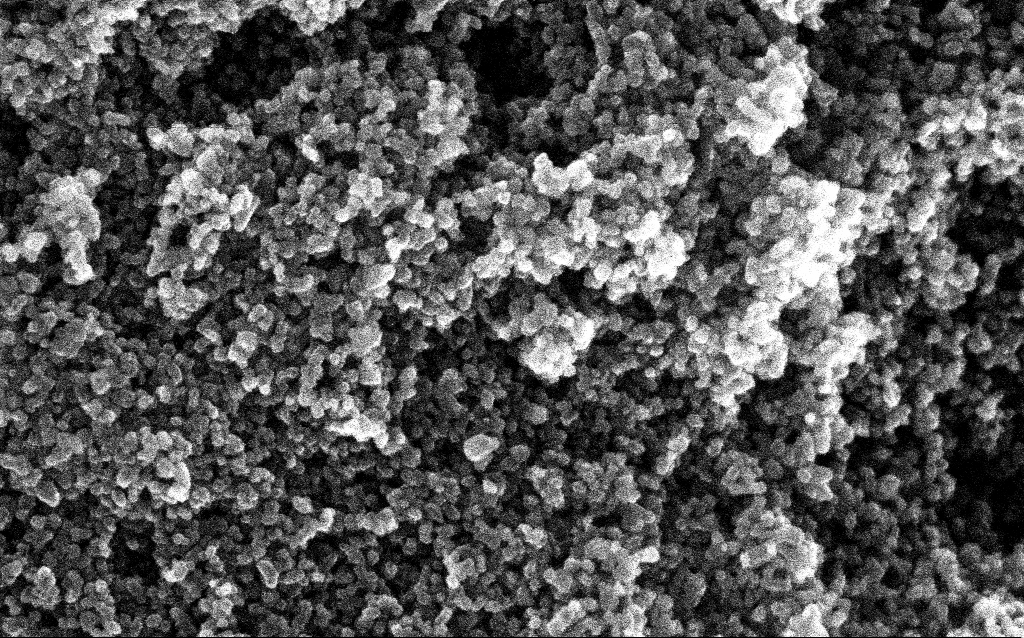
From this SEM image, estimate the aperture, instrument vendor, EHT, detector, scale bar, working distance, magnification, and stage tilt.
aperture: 30 µm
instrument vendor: Zeiss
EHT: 10 kV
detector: InLens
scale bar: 100 nm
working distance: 2.8 mm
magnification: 211.33 K X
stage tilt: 0°